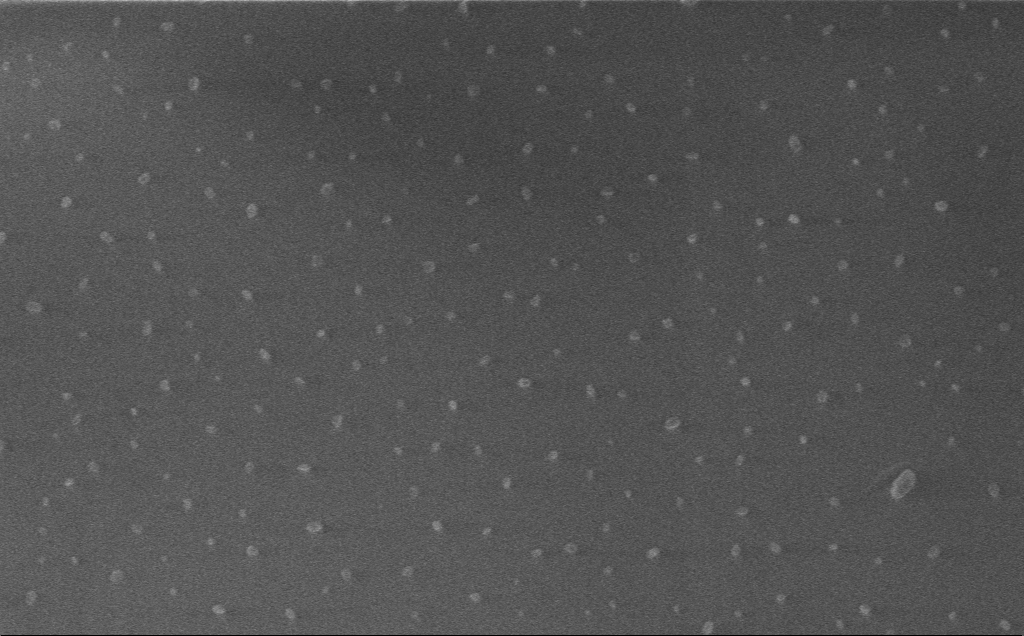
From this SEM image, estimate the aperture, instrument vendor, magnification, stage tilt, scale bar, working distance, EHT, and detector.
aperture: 30 µm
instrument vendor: Zeiss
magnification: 52.02 K X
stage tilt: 0°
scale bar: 1000 nm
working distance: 3 mm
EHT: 1 kV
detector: InLens